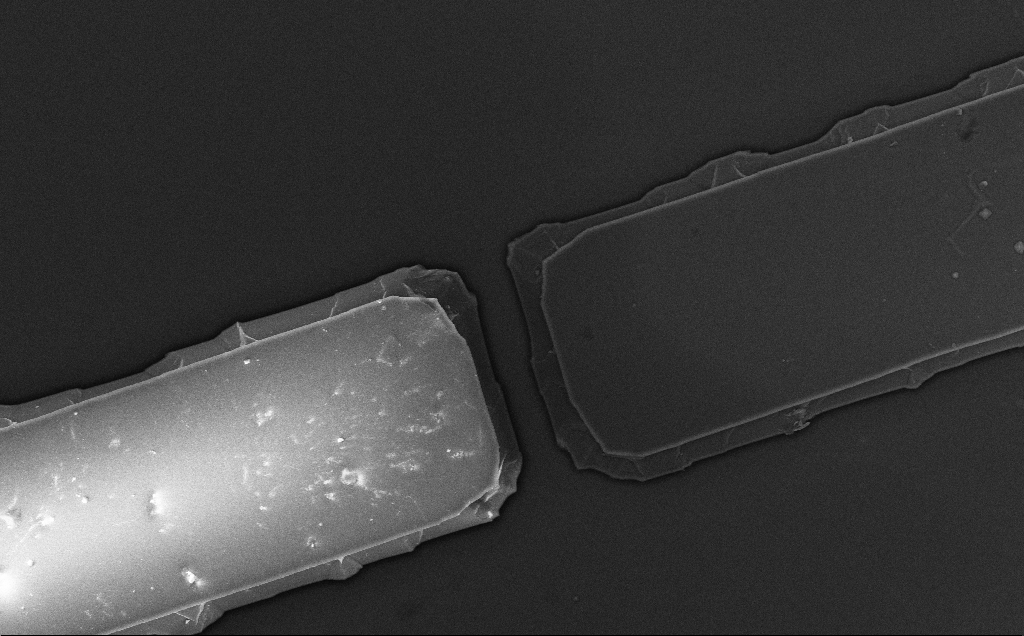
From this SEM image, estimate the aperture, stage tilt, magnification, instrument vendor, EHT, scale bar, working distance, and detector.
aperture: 30 µm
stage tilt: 0°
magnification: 5.5 K X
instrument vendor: Zeiss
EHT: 5 kV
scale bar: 10000 nm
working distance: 10 mm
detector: InLens